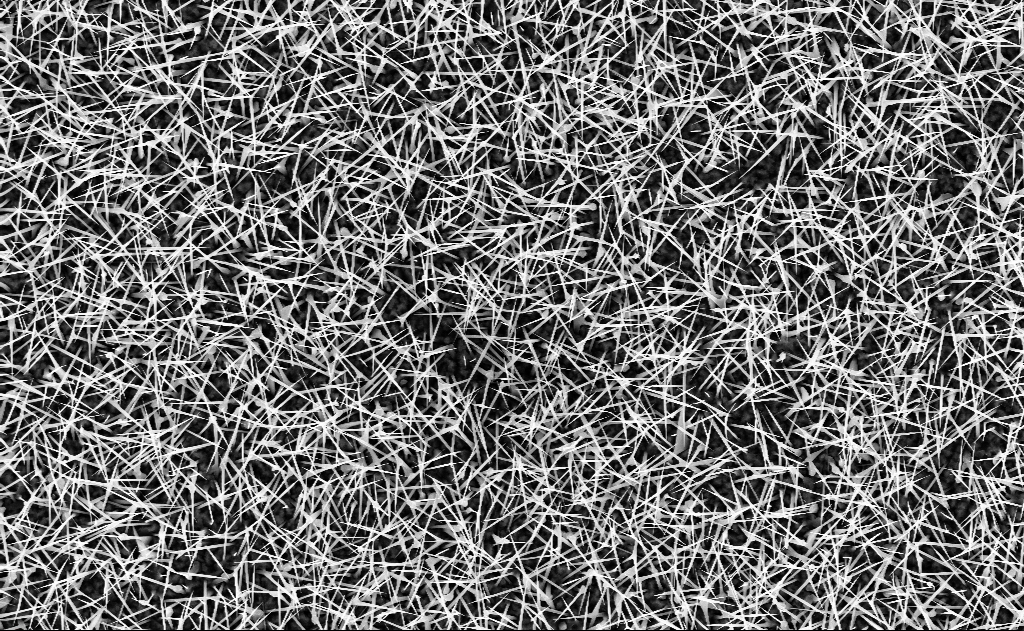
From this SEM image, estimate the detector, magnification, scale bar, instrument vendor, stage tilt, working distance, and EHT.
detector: InLens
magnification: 10 K X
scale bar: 2000 nm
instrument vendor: Zeiss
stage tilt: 0°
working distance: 9 mm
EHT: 10 kV